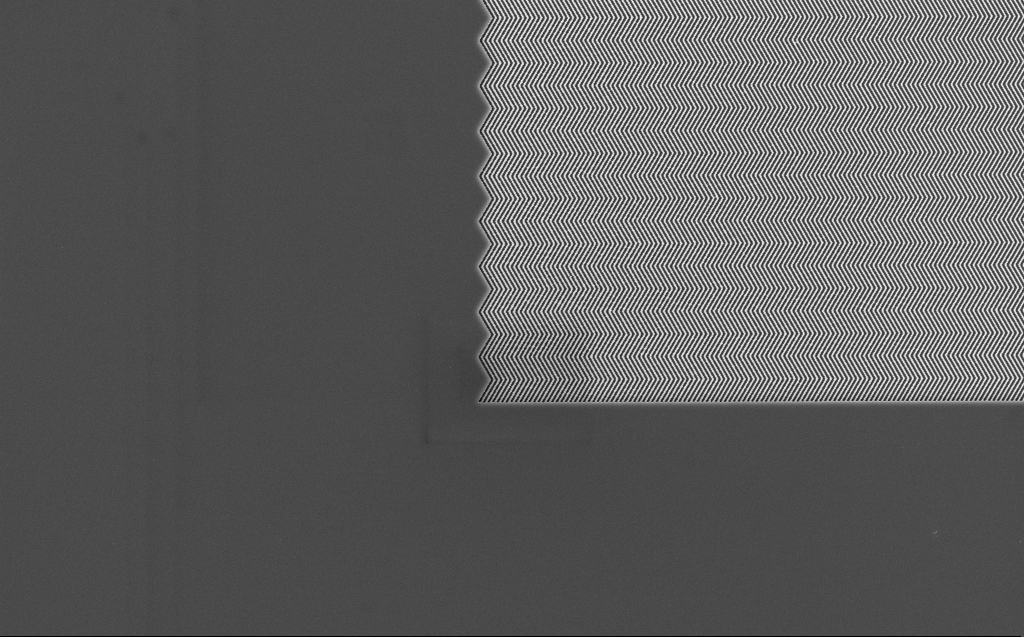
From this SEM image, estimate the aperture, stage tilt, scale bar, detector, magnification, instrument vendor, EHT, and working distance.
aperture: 30 µm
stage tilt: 0°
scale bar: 2000 nm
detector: InLens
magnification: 8.36 K X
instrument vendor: Zeiss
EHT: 5 kV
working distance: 7 mm